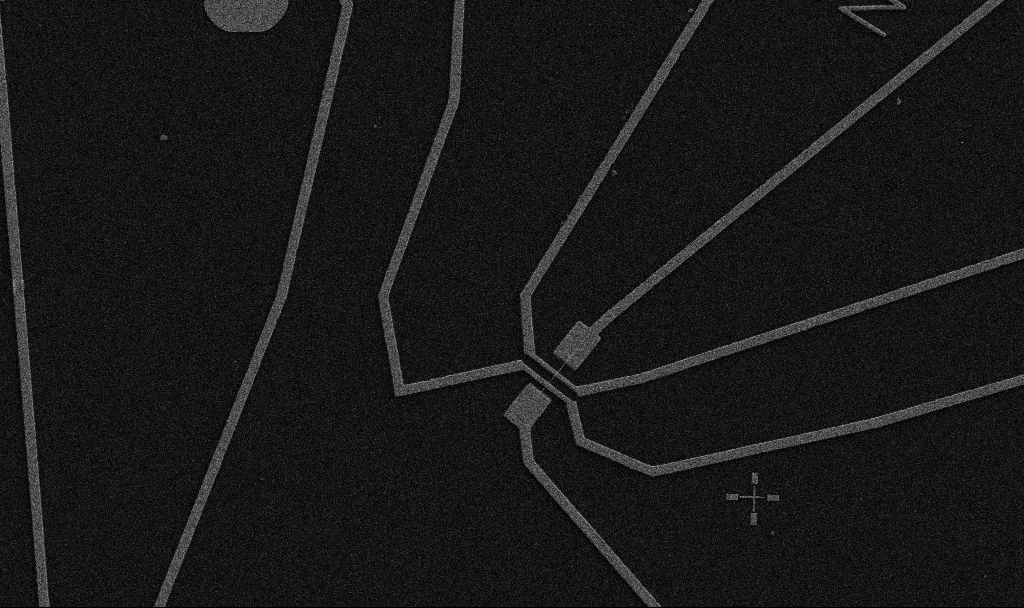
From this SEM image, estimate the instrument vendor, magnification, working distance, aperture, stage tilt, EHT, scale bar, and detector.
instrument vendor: Zeiss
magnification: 5 K X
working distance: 10.7 mm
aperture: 30 µm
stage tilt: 0°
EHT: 5 kV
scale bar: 10000 nm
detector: SE2